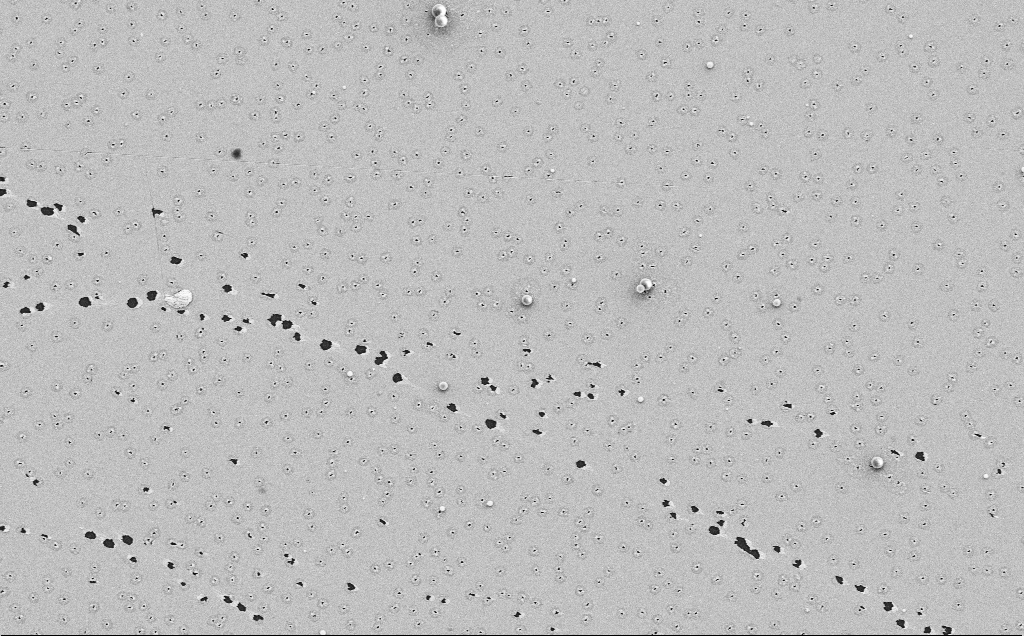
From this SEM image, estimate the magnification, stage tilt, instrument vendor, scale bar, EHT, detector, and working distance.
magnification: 4.2 K X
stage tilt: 0°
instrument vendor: Zeiss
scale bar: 10000 nm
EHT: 5 kV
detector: SE2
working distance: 12 mm